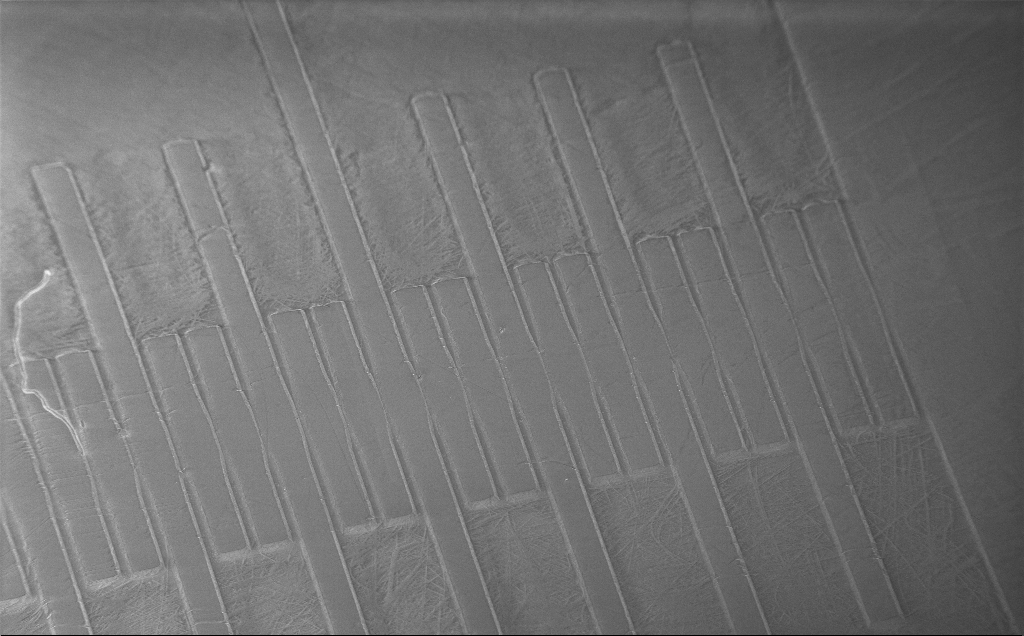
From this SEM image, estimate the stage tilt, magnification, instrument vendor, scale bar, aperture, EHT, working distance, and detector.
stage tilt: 45°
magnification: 0.145 K X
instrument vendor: Zeiss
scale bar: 100000 nm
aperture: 30 µm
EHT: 3 kV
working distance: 9 mm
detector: InLens